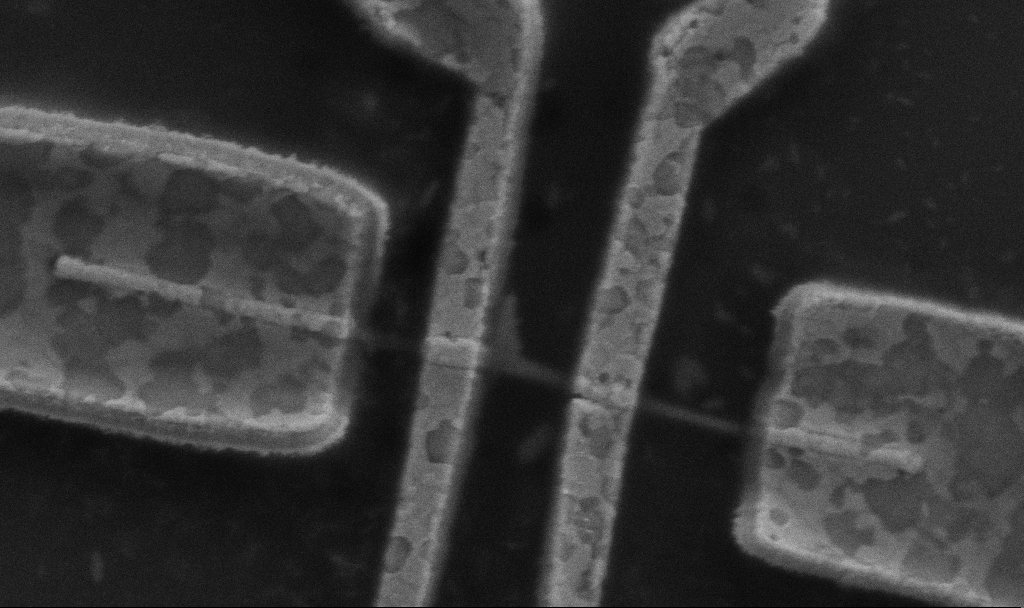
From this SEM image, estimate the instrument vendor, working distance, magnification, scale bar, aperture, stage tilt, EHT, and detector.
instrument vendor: Zeiss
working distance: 9.7 mm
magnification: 52.05 K X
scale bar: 1000 nm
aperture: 30 µm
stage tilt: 0°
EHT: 5 kV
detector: SE2